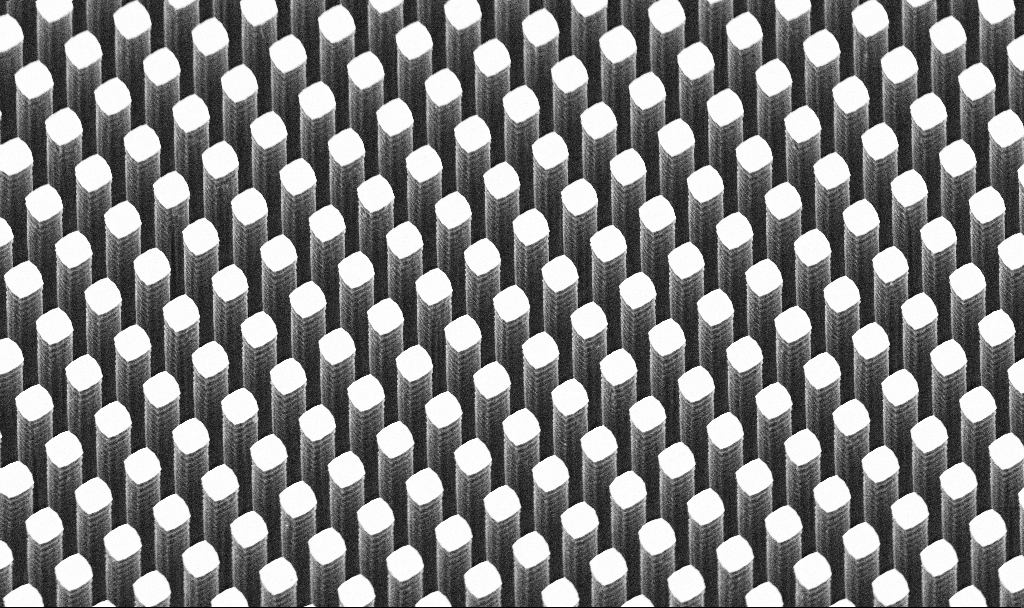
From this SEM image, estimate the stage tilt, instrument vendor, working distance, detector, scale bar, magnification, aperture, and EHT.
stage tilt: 45°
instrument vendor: Zeiss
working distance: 7.5 mm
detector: SE2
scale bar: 10000 nm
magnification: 5.24 K X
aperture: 30 µm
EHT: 5 kV